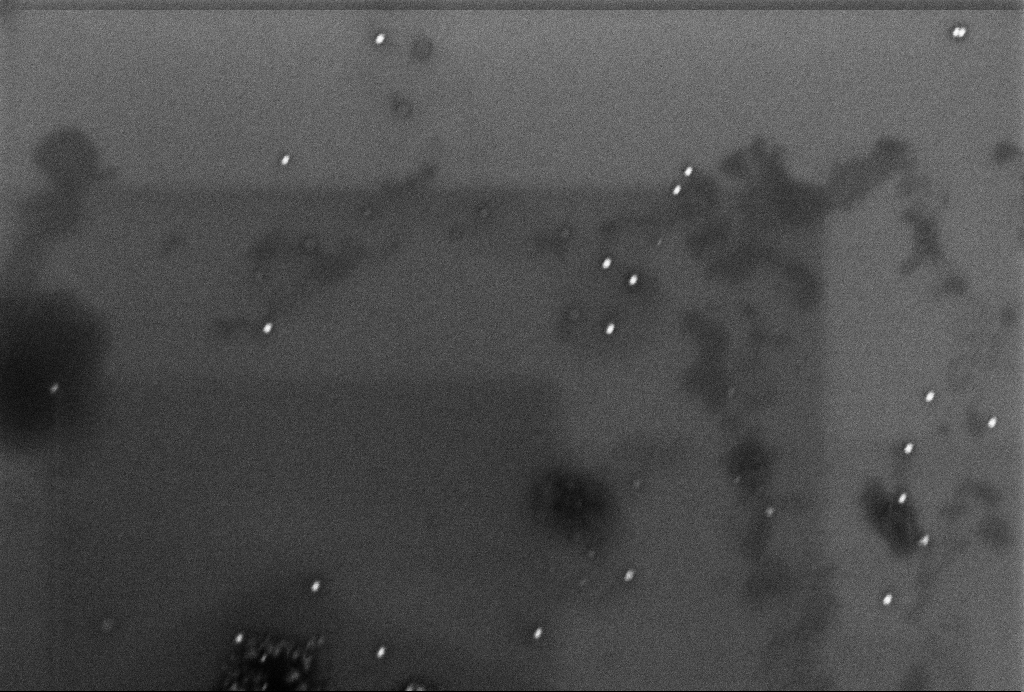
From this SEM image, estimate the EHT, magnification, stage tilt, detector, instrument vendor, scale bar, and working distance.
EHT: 2 kV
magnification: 85 K X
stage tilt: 0°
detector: InLens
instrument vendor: Zeiss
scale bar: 200 nm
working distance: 3.3 mm